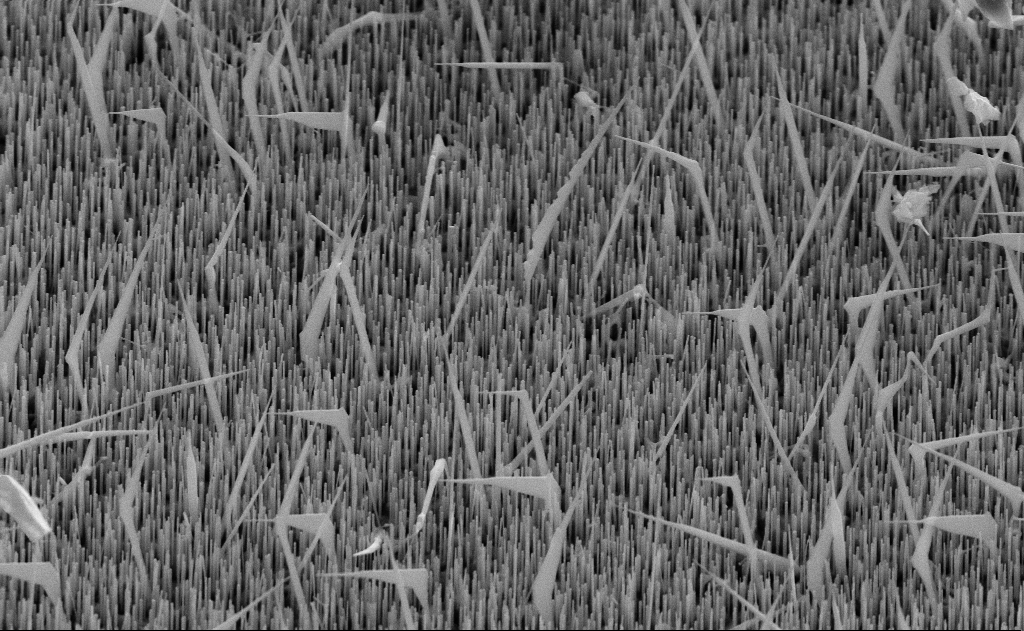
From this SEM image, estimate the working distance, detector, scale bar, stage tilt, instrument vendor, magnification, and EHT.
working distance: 11 mm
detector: SE2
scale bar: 1000 nm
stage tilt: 45°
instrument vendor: Zeiss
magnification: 20 K X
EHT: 10 kV